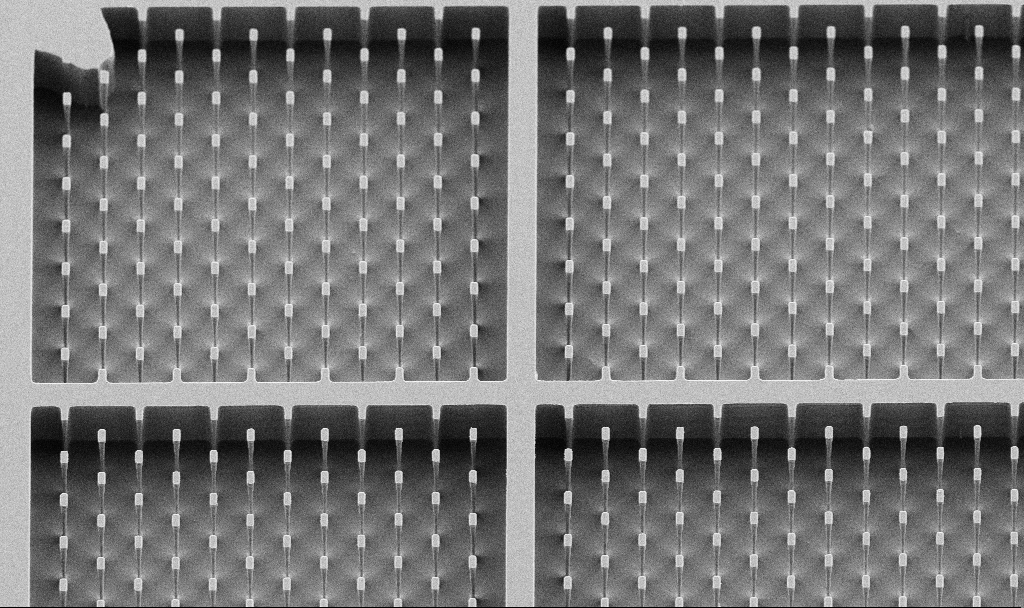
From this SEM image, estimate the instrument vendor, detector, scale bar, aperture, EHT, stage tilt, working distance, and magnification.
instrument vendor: Zeiss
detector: SE2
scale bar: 10000 nm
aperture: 30 µm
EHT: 5 kV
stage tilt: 30°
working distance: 6.8 mm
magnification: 1.52 K X